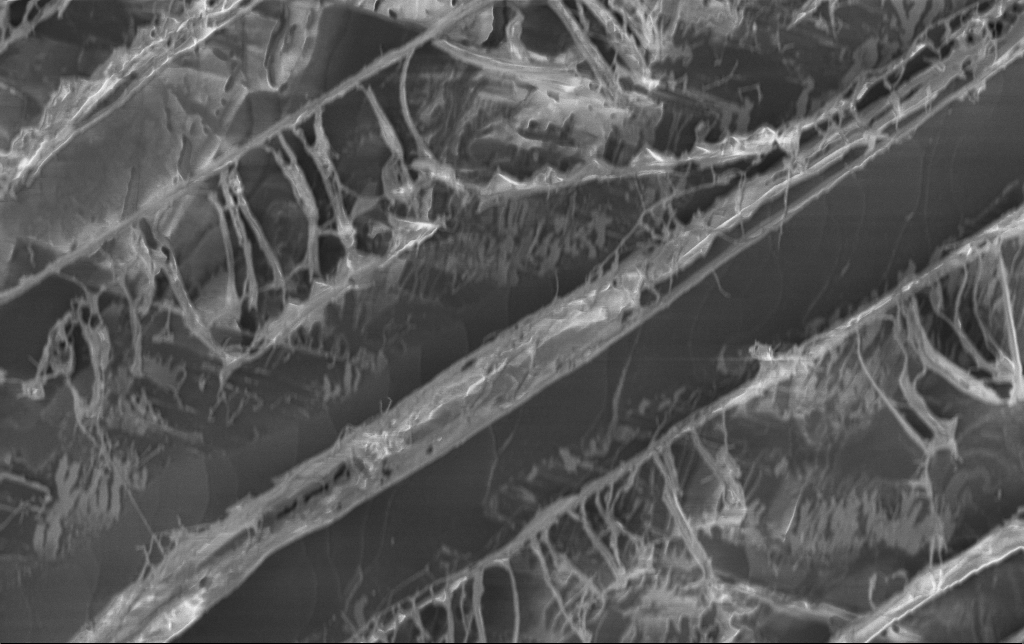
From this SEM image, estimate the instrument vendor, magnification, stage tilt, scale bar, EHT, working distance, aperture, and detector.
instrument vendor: Zeiss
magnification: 4.35 K X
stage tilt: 0°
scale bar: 10000 nm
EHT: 1.5 kV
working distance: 3.1 mm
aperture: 30 µm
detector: InLens